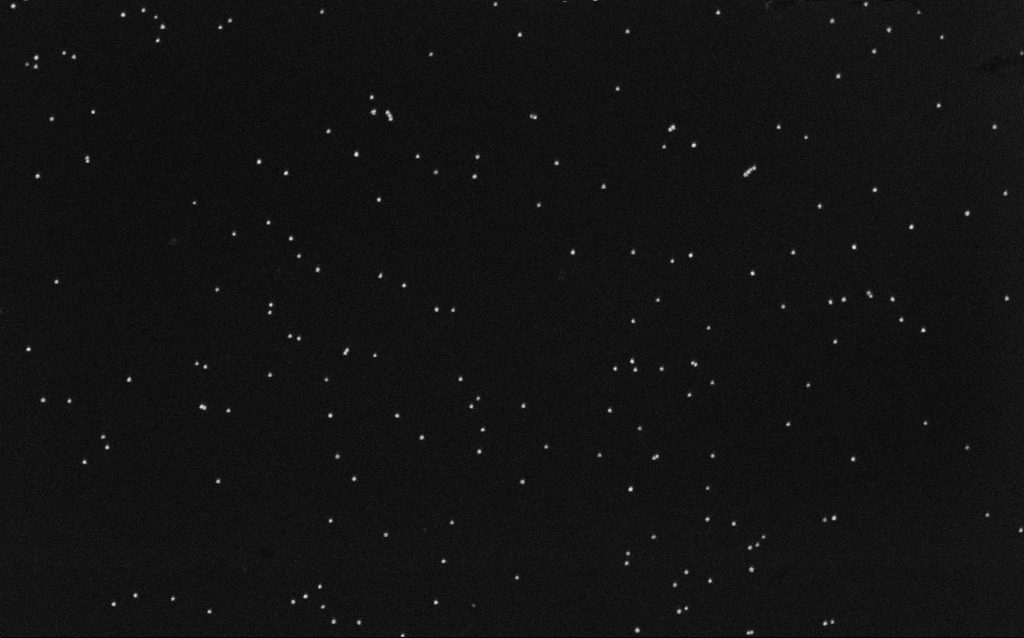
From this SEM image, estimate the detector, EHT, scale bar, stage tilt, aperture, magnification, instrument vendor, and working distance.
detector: InLens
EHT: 10 kV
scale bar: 200 nm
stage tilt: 0°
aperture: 30 µm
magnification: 100 K X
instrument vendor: Zeiss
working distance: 6.5 mm